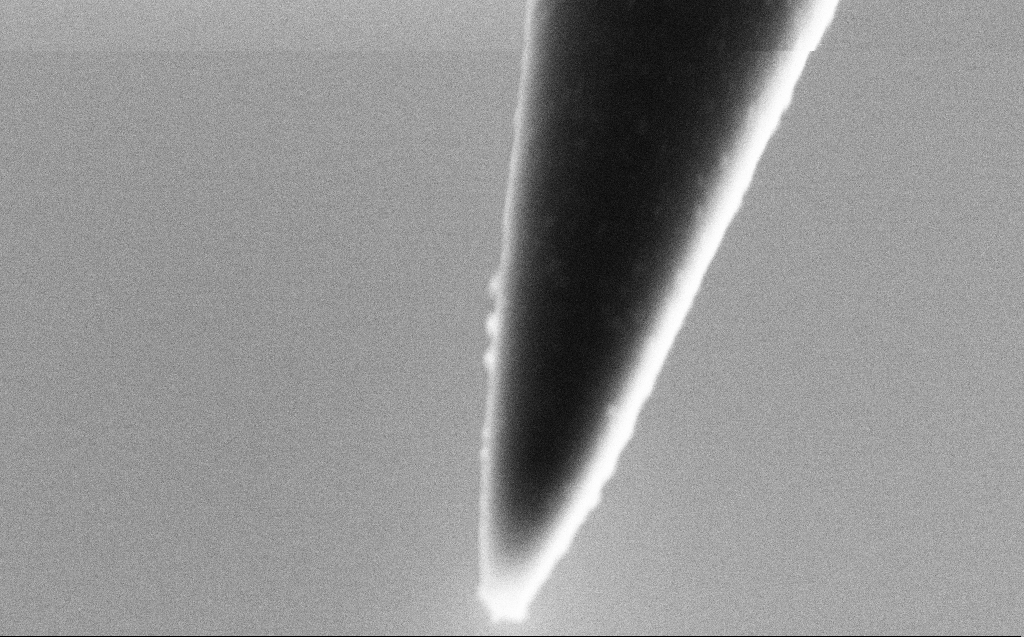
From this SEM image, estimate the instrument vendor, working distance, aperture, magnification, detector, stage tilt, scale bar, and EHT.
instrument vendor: Zeiss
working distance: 3 mm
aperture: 30 µm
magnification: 151.54 K X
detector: SE2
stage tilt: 45°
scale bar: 200 nm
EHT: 3 kV